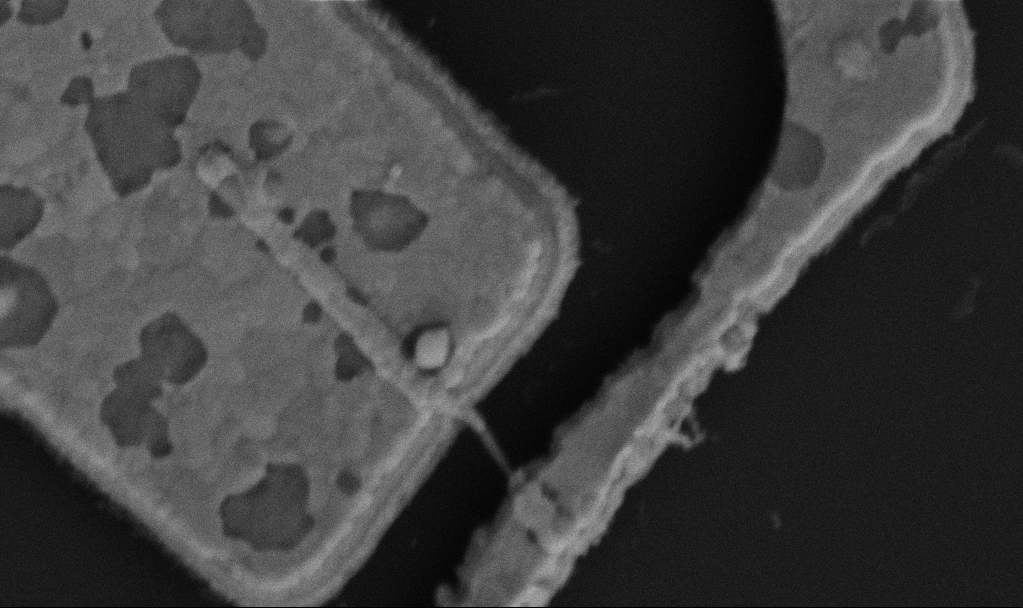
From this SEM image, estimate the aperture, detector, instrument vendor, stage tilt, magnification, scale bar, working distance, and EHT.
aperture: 30 µm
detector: SE2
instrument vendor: Zeiss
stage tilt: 0°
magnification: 80 K X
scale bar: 200 nm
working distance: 10.4 mm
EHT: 5 kV